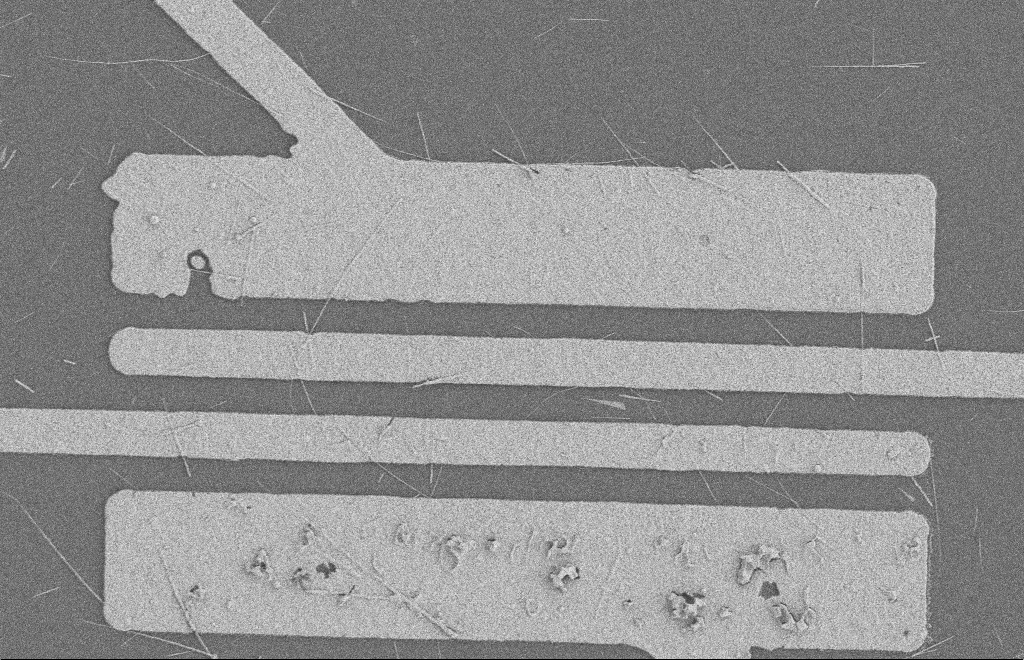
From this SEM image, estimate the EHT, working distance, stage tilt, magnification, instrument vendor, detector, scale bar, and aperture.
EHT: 2 kV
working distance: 8 mm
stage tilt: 0°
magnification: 4.96 K X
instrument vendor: Zeiss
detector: SE2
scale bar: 2000 nm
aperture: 20 µm